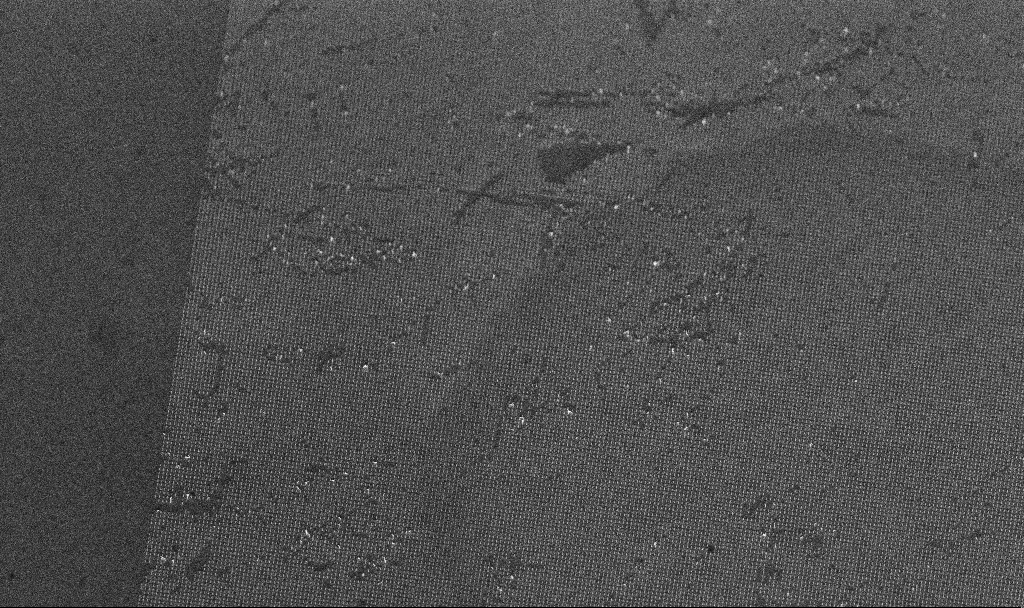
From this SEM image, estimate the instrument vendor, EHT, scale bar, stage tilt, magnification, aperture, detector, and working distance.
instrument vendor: Zeiss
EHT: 5 kV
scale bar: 10000 nm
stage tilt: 45°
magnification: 6.34 K X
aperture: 30 µm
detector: InLens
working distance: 7.4 mm